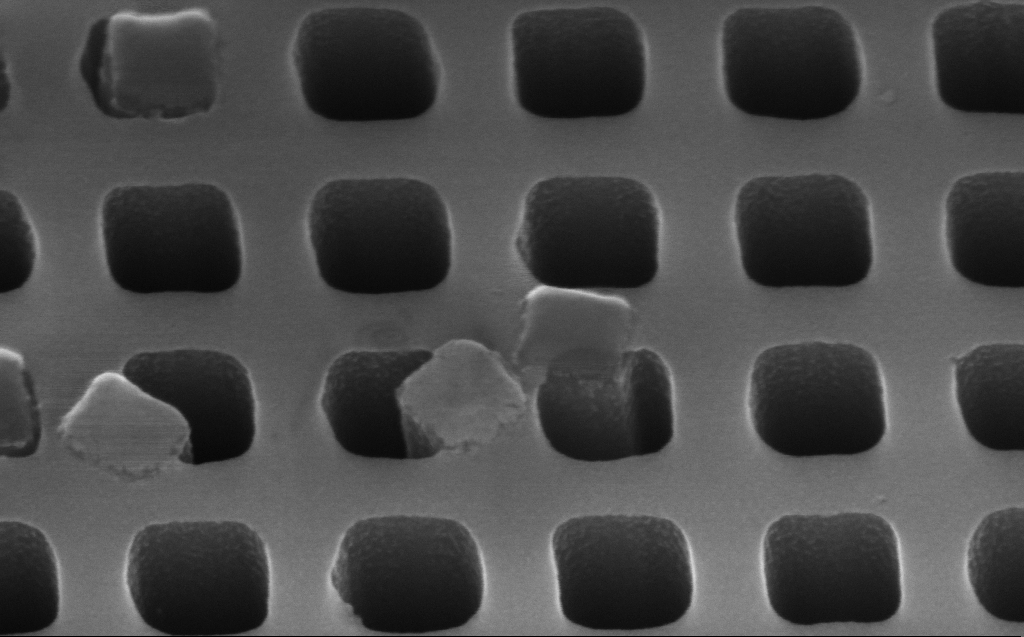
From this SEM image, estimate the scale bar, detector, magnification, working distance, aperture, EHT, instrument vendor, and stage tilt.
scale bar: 200 nm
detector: InLens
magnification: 156.49 K X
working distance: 7 mm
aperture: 30 µm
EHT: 10 kV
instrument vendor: Zeiss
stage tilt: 45°